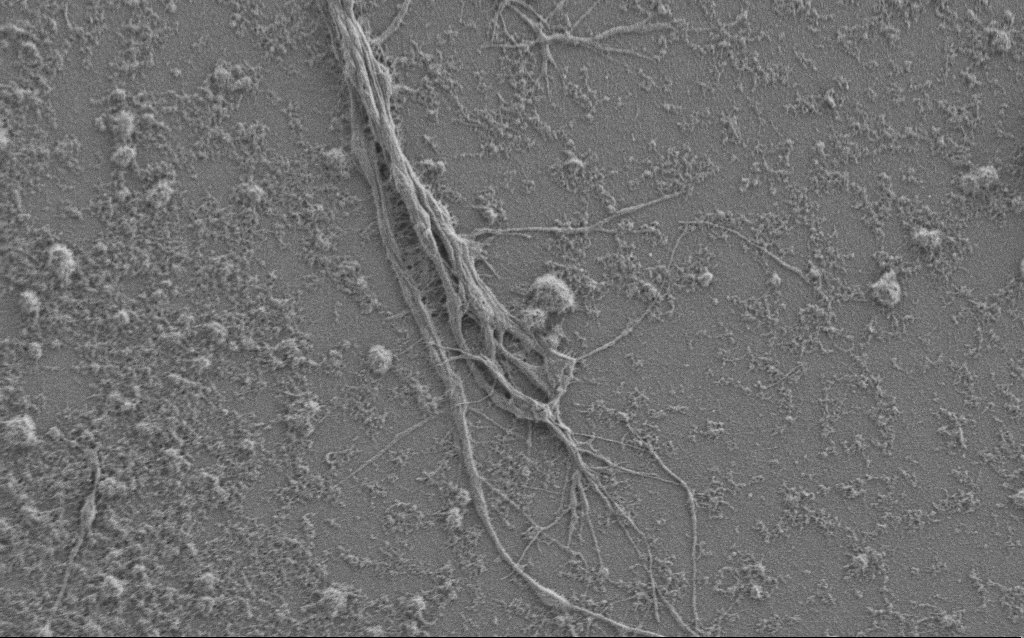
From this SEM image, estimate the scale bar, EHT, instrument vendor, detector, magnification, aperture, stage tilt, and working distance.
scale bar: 10000 nm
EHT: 1 kV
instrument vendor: Zeiss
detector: SE2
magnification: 6 K X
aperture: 30 µm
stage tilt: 0°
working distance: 6 mm